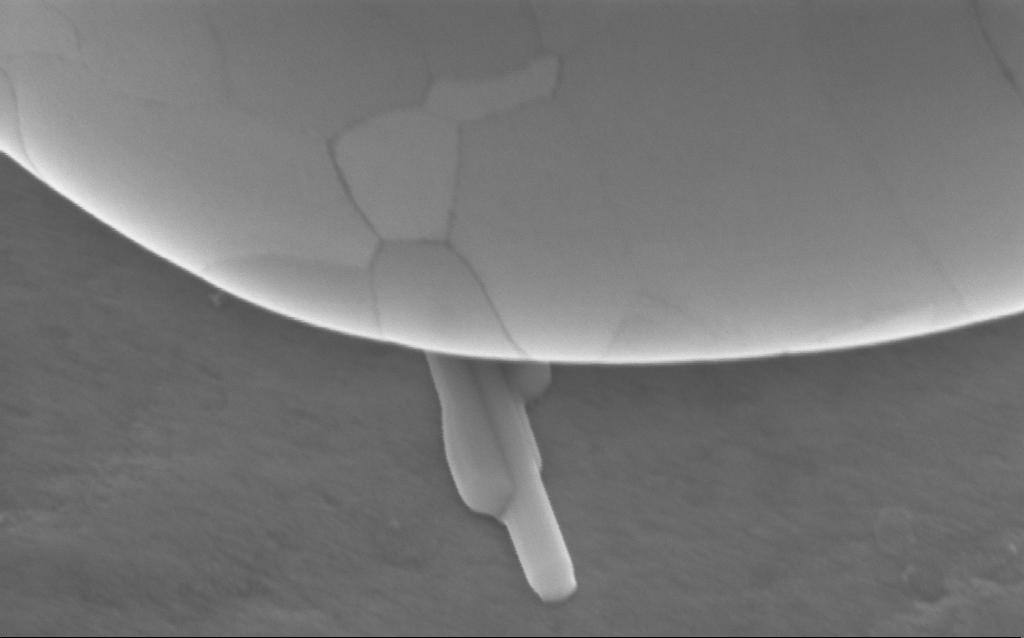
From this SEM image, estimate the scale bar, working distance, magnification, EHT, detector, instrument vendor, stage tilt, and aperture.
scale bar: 200 nm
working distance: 4 mm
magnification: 179.14 K X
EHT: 5 kV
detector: InLens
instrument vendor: Zeiss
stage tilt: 35°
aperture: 30 µm